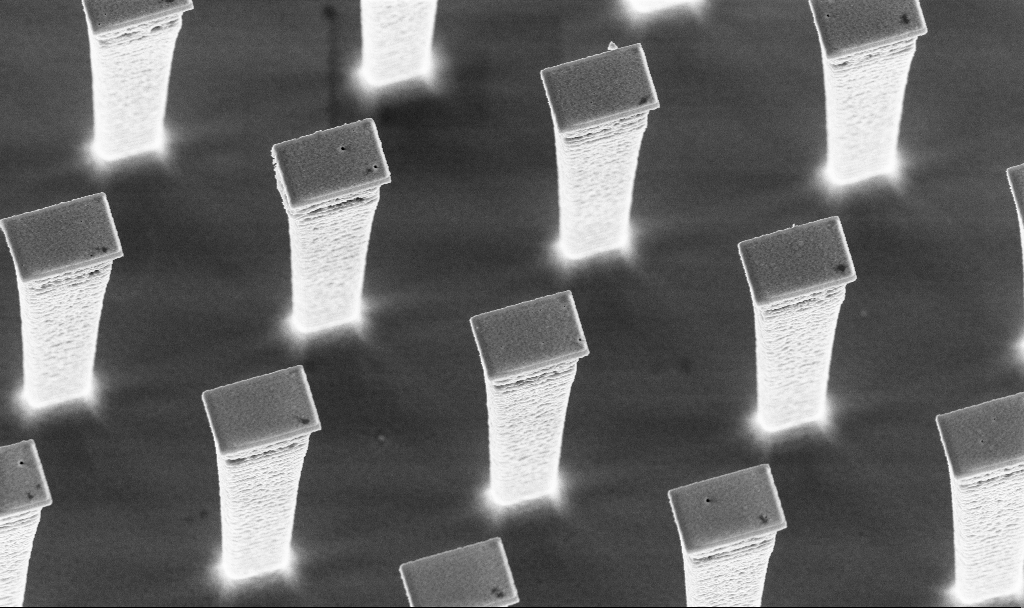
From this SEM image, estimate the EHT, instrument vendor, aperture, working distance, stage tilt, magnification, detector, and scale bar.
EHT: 5 kV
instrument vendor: Zeiss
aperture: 30 µm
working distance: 3 mm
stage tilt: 20°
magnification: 8.6 K X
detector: InLens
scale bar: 2000 nm